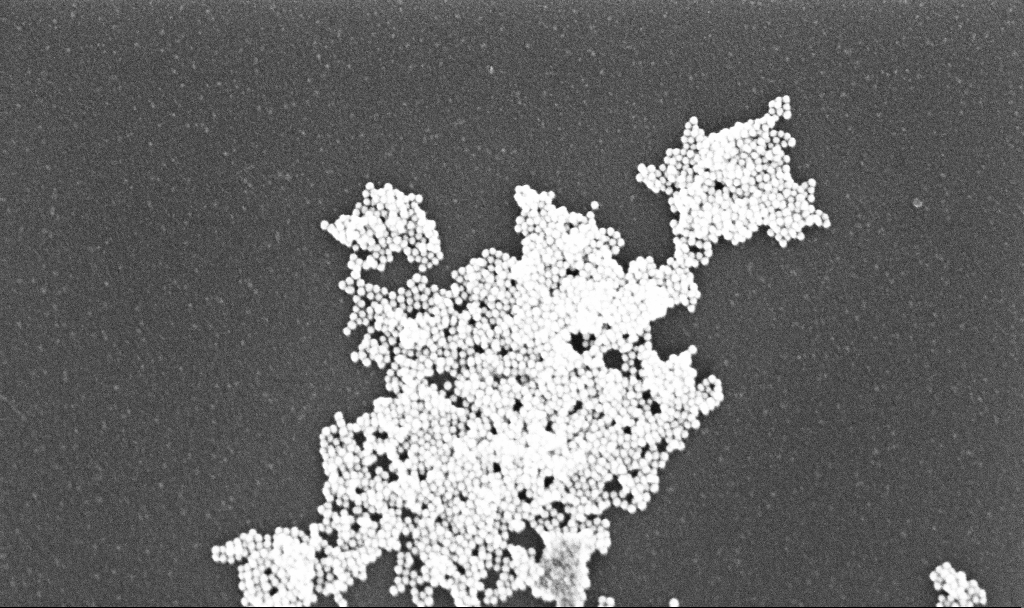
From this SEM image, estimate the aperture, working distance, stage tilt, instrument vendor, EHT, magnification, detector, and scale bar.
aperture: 30 µm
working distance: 3.1 mm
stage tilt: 0°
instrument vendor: Zeiss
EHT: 10 kV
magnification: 122.95 K X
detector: InLens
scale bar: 200 nm